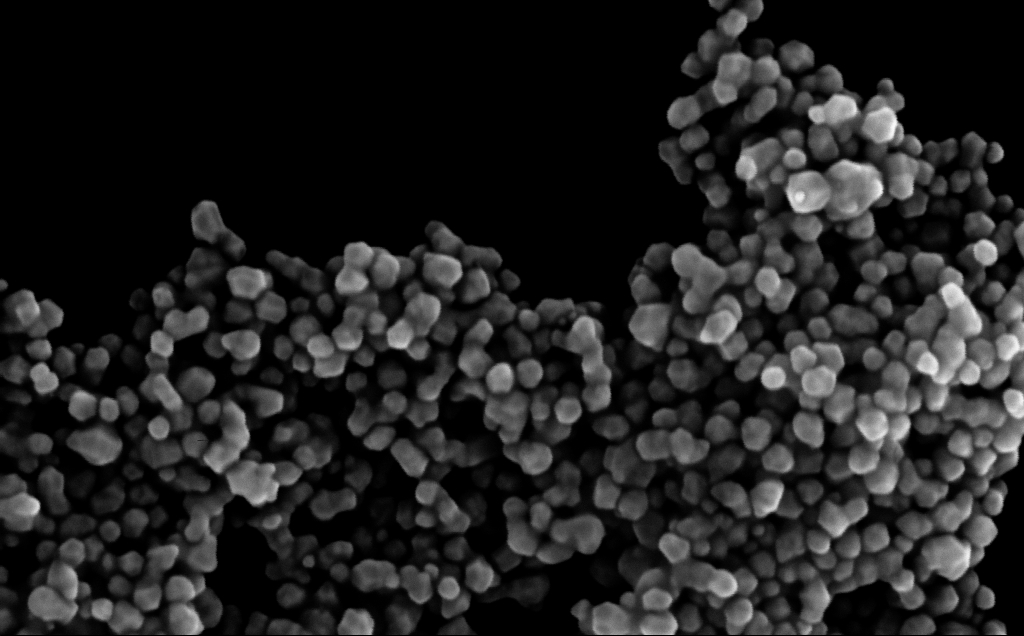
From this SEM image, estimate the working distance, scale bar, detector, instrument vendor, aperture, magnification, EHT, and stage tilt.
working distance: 3 mm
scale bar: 200 nm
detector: InLens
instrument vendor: Zeiss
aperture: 30 µm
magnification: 266.8 K X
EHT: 10 kV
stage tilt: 0°